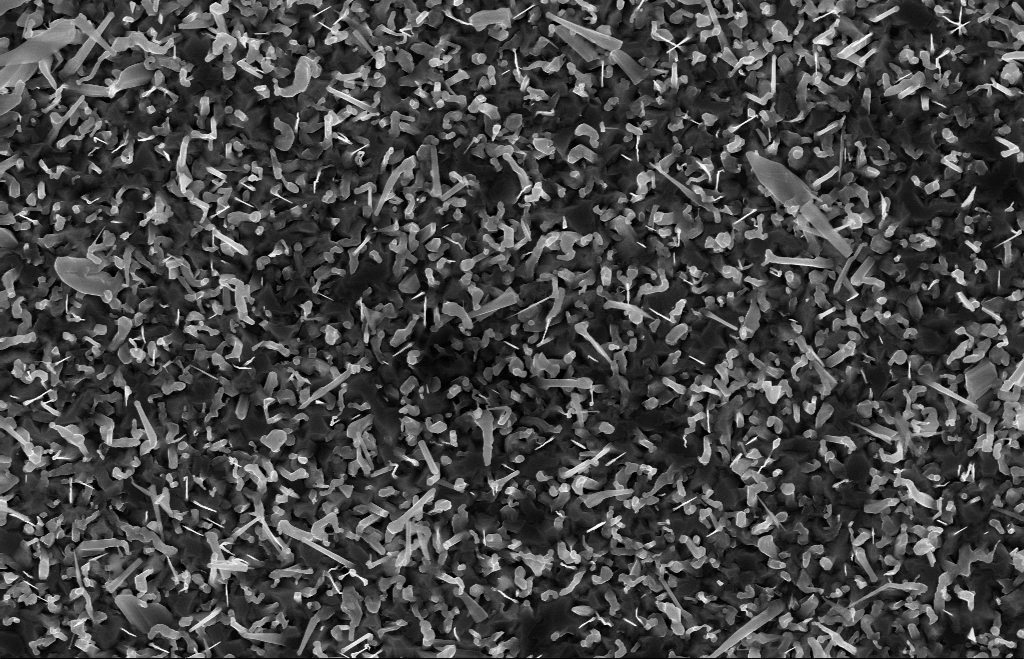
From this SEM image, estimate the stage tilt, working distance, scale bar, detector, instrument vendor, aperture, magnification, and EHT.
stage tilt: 0°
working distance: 9 mm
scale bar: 1000 nm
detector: InLens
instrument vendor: Zeiss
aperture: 30 µm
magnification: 20 K X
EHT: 10 kV